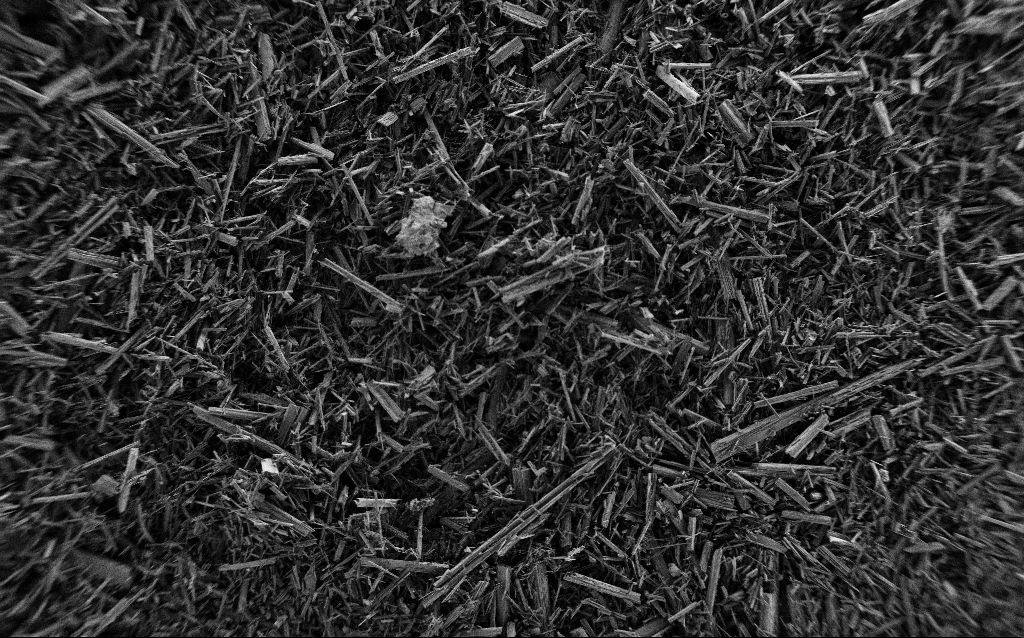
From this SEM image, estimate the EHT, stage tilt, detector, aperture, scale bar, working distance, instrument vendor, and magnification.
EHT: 0.8 kV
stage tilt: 0°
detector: SE2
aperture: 30 µm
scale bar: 200000 nm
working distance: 4.5 mm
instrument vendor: Zeiss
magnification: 0.25 K X